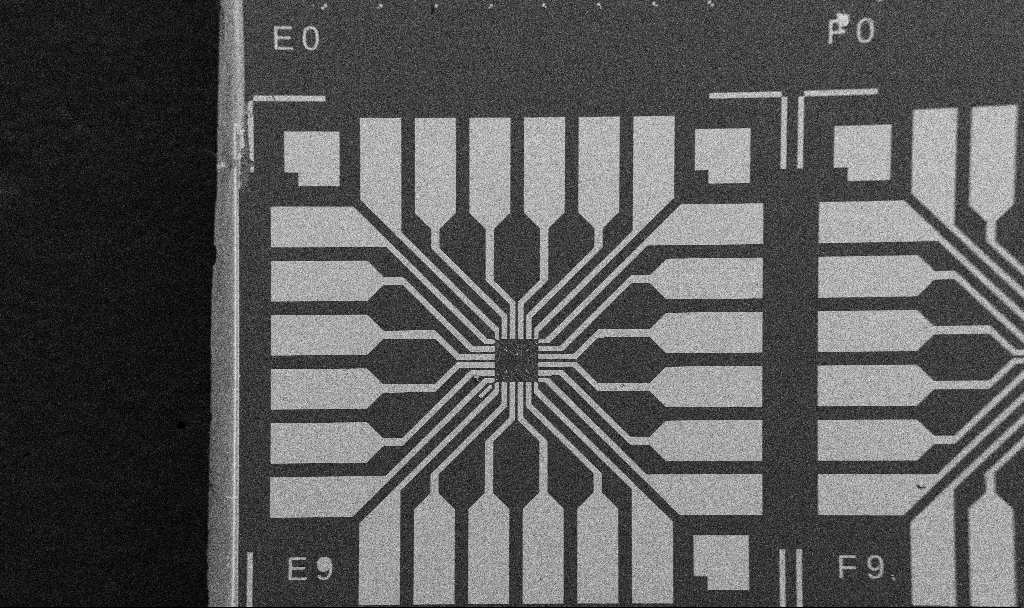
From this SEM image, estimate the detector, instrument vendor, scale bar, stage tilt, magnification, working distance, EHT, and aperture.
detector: SE2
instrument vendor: Zeiss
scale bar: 200000 nm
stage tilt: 0°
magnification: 0.1 K X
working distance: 10.7 mm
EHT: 5 kV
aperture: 30 µm